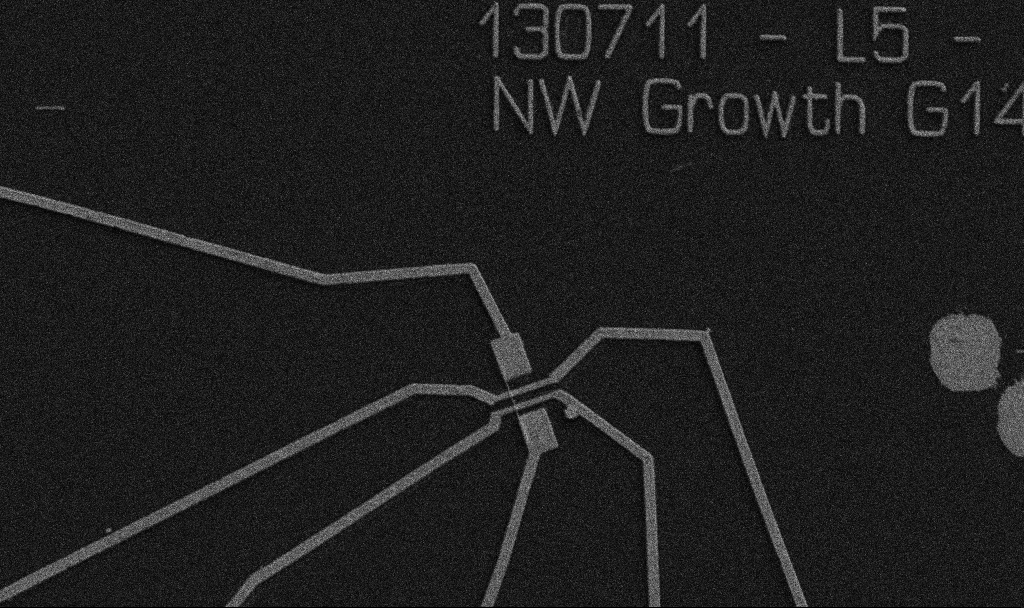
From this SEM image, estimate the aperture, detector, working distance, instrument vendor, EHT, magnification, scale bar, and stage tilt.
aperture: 30 µm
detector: SE2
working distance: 10.7 mm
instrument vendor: Zeiss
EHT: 5 kV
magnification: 5 K X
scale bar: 10000 nm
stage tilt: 0°